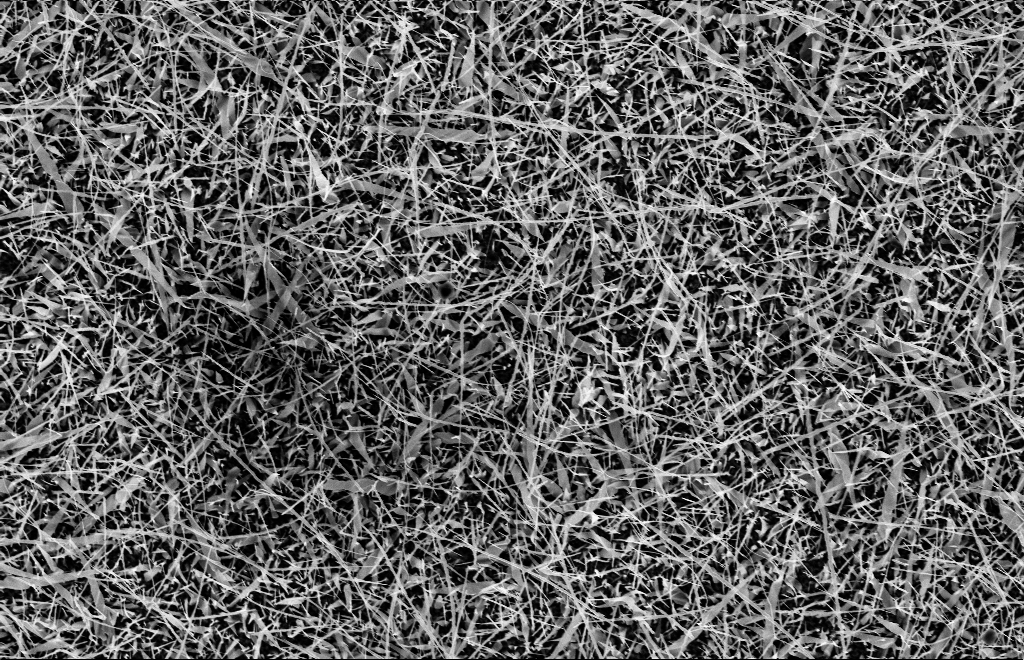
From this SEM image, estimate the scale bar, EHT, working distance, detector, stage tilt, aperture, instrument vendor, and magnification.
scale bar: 2000 nm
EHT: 10 kV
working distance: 15 mm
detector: InLens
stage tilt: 0°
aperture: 30 µm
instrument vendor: Zeiss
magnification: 5 K X